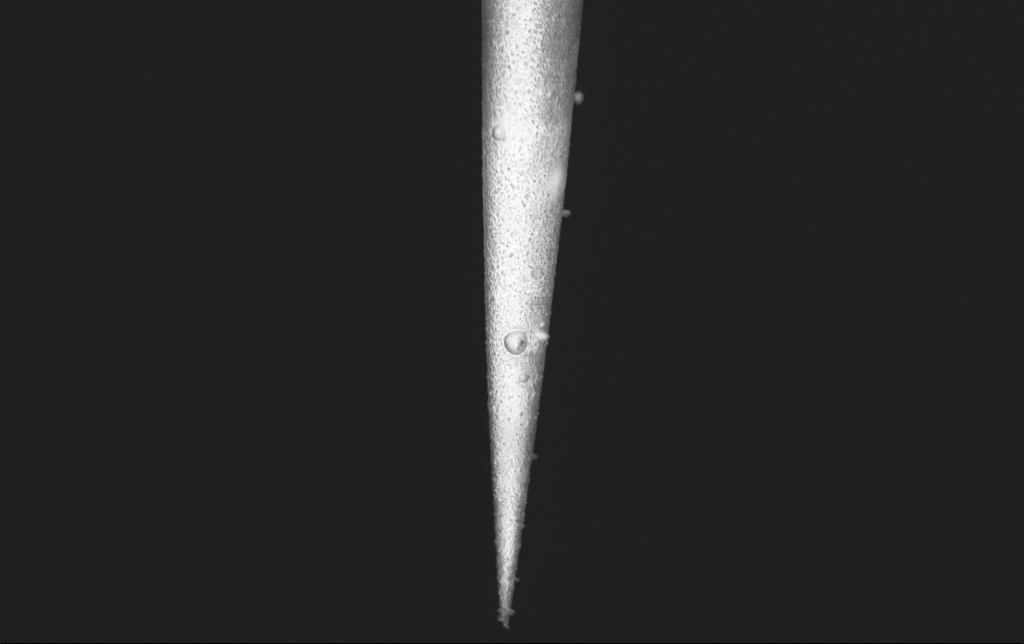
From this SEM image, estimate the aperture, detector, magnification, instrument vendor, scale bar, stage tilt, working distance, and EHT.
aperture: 30 µm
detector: InLens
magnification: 25 K X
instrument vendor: Zeiss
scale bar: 2000 nm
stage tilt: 0°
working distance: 6 mm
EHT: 2 kV